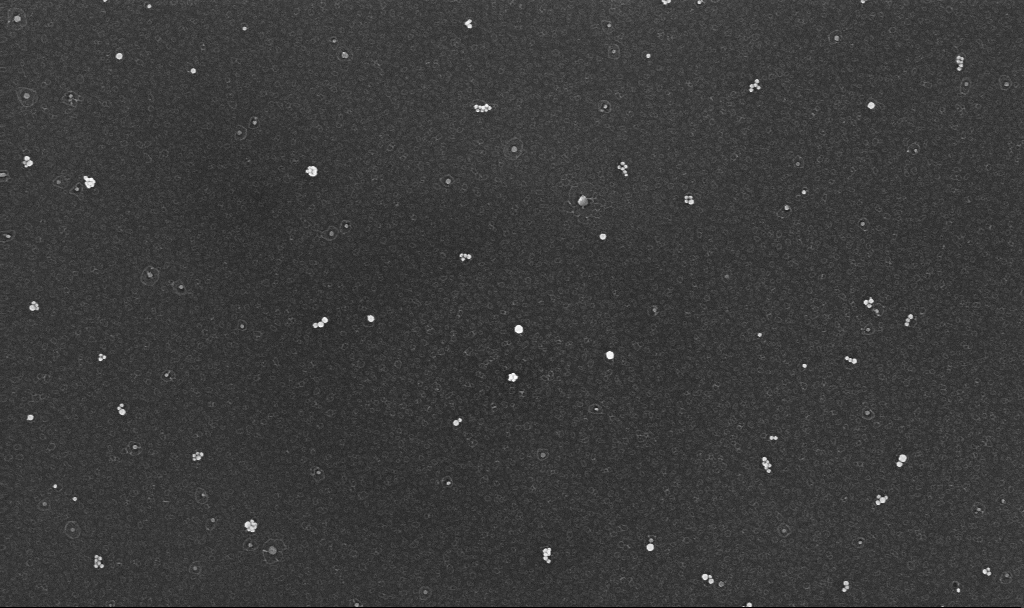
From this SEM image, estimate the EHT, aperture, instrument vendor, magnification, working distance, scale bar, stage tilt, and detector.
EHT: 10 kV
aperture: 30 µm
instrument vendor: Zeiss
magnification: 70 K X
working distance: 3.4 mm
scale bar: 1000 nm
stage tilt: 0°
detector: InLens